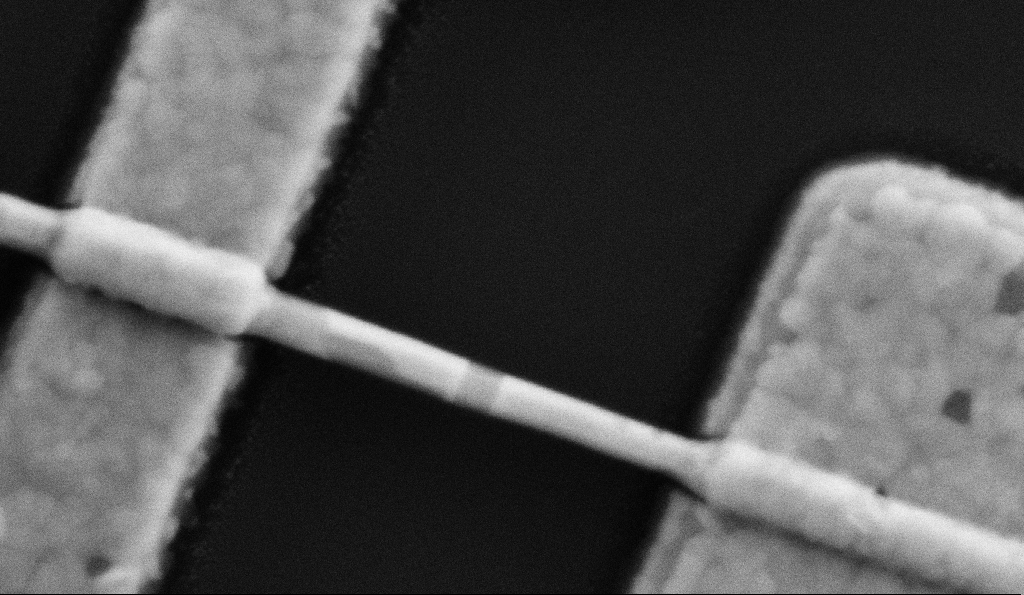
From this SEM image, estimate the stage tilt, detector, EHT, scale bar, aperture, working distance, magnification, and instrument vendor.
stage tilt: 0°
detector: SE2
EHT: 5 kV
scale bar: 200 nm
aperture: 30 µm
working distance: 8.5 mm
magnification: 200 K X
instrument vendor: Zeiss